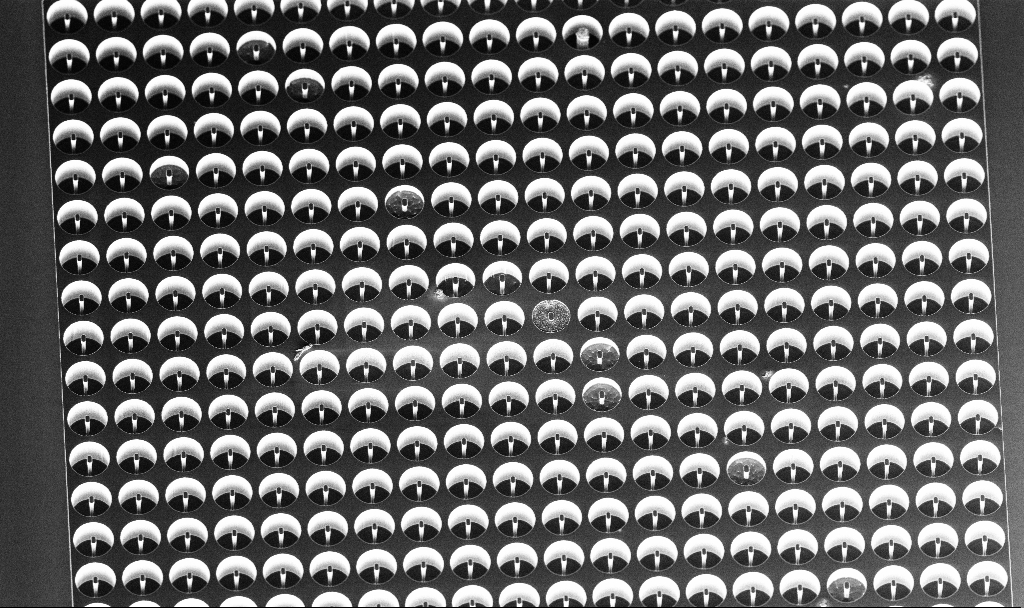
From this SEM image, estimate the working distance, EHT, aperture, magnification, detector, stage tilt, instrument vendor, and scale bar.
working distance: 6.8 mm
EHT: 5 kV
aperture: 30 µm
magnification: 0.748 K X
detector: InLens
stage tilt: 29.2°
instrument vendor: Zeiss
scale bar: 20000 nm